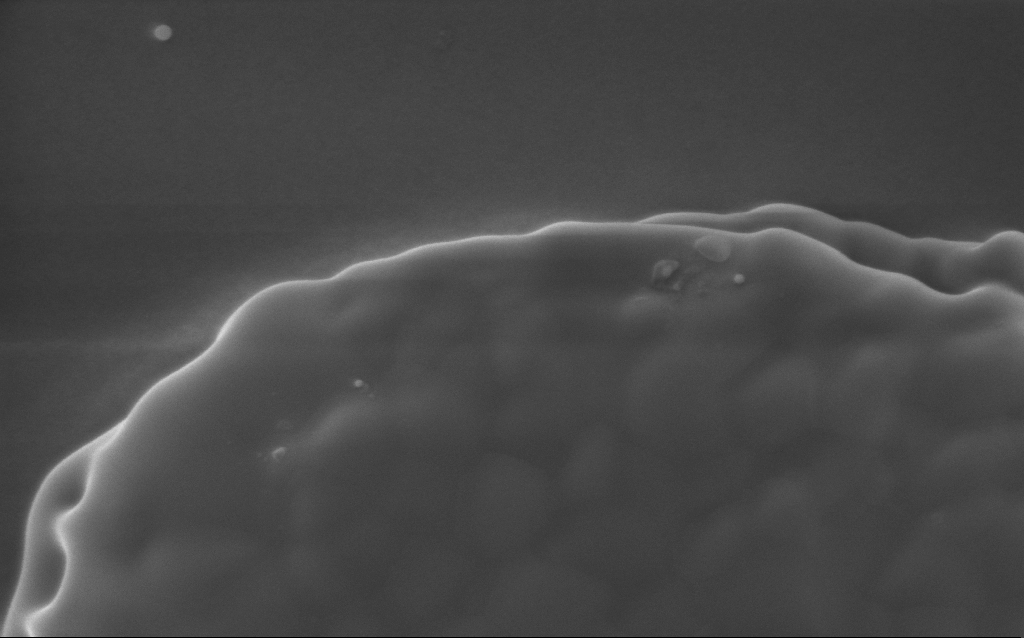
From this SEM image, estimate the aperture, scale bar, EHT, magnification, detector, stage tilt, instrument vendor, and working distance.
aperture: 30 µm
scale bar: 200 nm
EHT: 5 kV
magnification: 172.38 K X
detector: InLens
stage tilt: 0°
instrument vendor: Zeiss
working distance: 3 mm